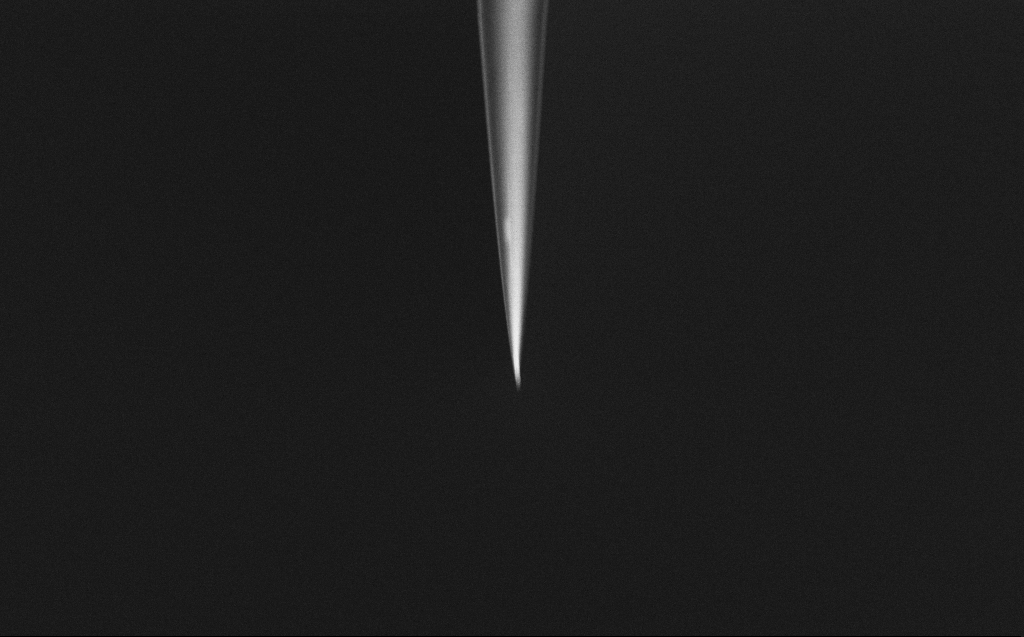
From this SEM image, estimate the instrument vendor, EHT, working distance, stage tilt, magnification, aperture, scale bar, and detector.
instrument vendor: Zeiss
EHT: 2 kV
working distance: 6 mm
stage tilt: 45°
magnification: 10 K X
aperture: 30 µm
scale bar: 2000 nm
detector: InLens